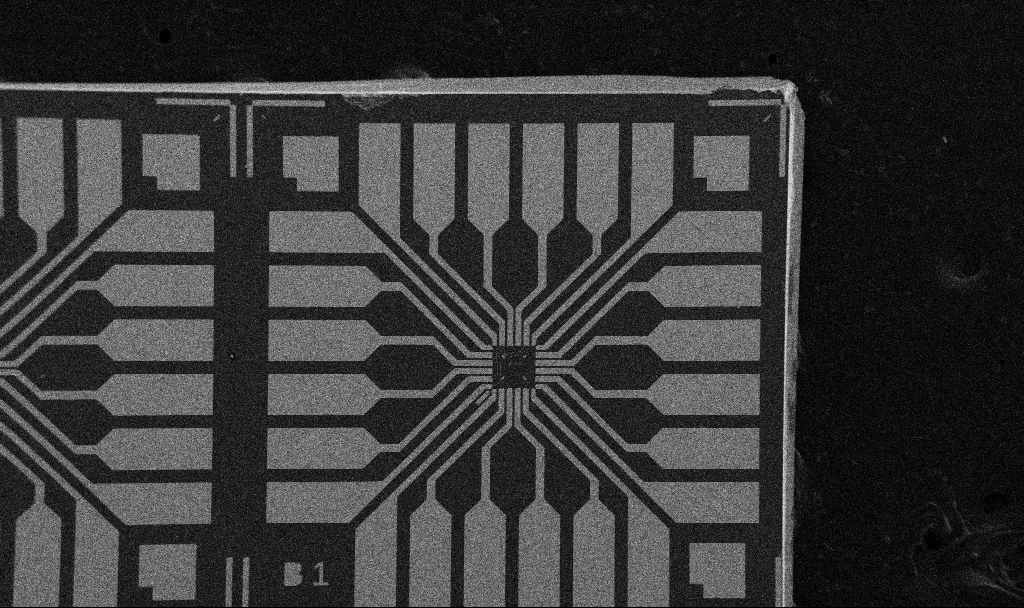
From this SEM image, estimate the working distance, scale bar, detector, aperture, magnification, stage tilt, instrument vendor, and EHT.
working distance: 10.7 mm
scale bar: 200000 nm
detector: SE2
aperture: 30 µm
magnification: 0.1 K X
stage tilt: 0°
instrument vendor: Zeiss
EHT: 5 kV